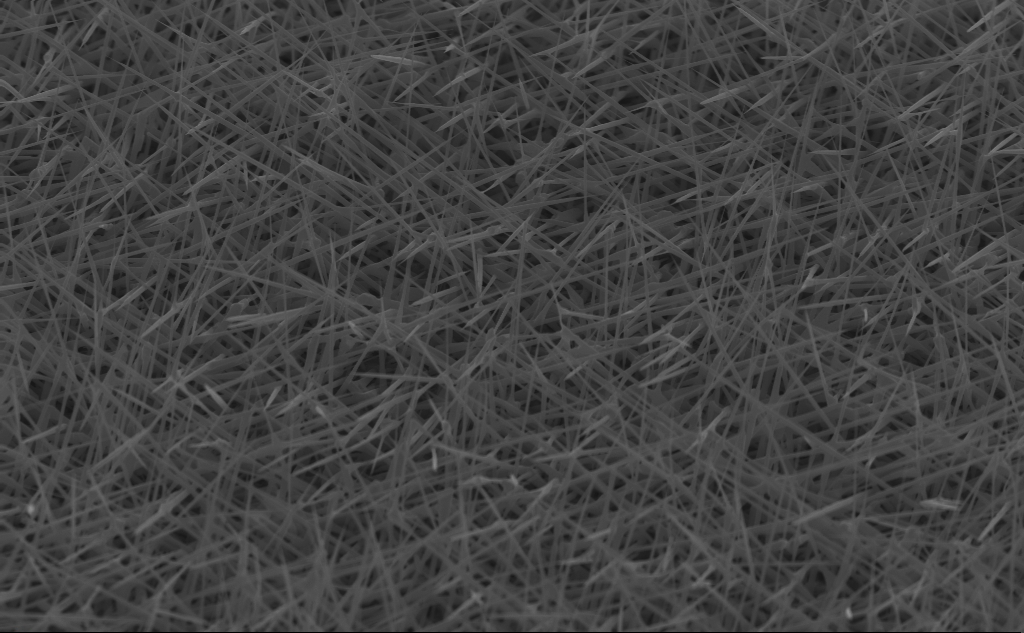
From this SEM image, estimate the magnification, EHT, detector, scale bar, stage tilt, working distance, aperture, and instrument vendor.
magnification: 20 K X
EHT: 10 kV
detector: InLens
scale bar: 2000 nm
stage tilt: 45°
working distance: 5 mm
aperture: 30 µm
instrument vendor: Zeiss